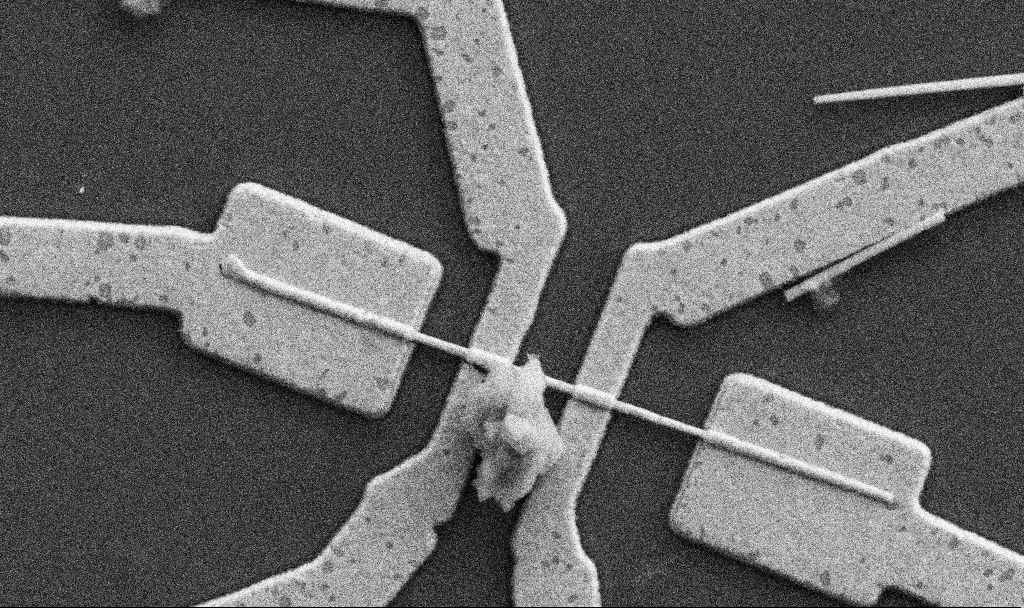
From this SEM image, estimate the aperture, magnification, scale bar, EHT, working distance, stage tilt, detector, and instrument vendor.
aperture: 30 µm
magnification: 40.64 K X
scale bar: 1000 nm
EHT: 5 kV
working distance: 9.8 mm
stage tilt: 0°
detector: SE2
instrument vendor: Zeiss